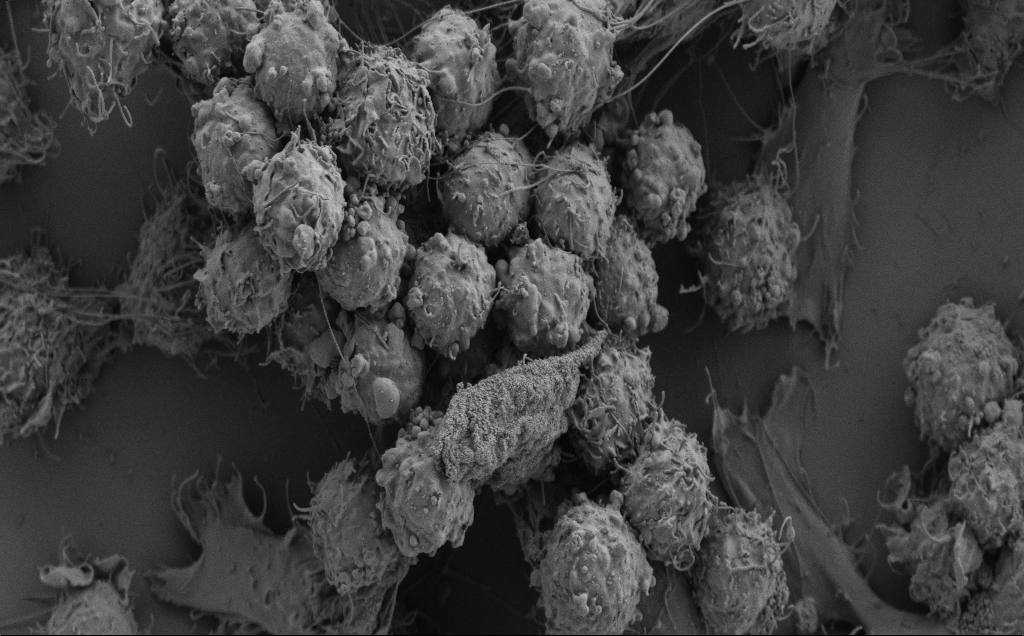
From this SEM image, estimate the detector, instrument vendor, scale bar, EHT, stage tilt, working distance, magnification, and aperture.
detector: SE2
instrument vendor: Zeiss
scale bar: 10000 nm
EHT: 3 kV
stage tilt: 0.8°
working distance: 6 mm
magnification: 4.54 K X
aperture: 30 µm